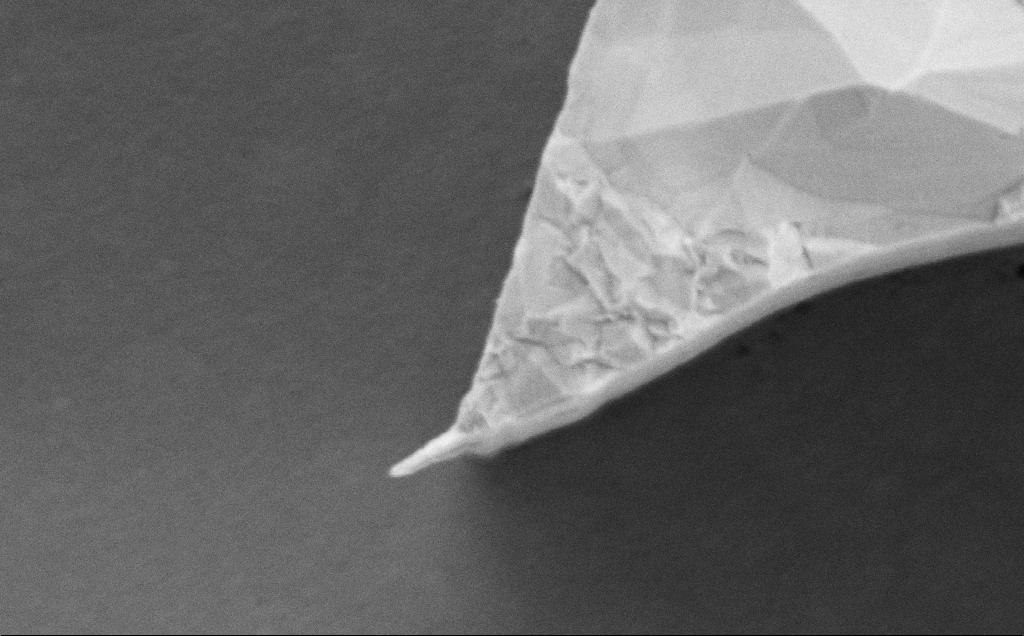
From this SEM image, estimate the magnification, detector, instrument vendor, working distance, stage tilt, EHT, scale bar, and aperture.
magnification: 63.21 K X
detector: SE2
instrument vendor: Zeiss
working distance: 13 mm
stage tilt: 0°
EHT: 5 kV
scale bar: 1000 nm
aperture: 30 µm